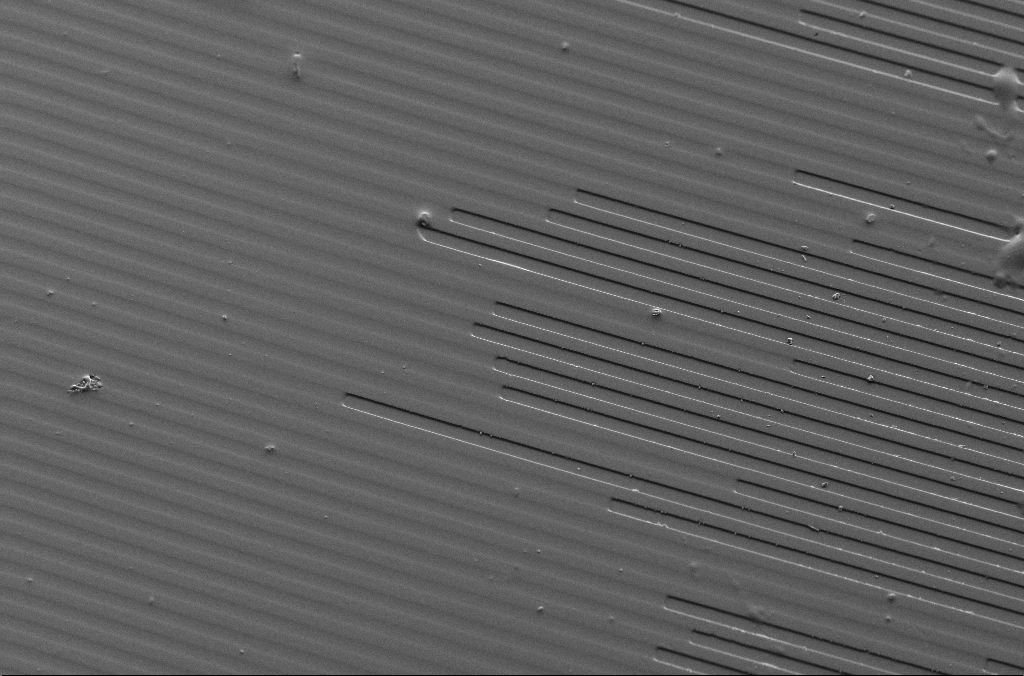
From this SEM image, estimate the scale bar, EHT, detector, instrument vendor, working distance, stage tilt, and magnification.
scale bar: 100000 nm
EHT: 5 kV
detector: SE2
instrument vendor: Zeiss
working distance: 7.2 mm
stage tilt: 40°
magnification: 0.5 K X